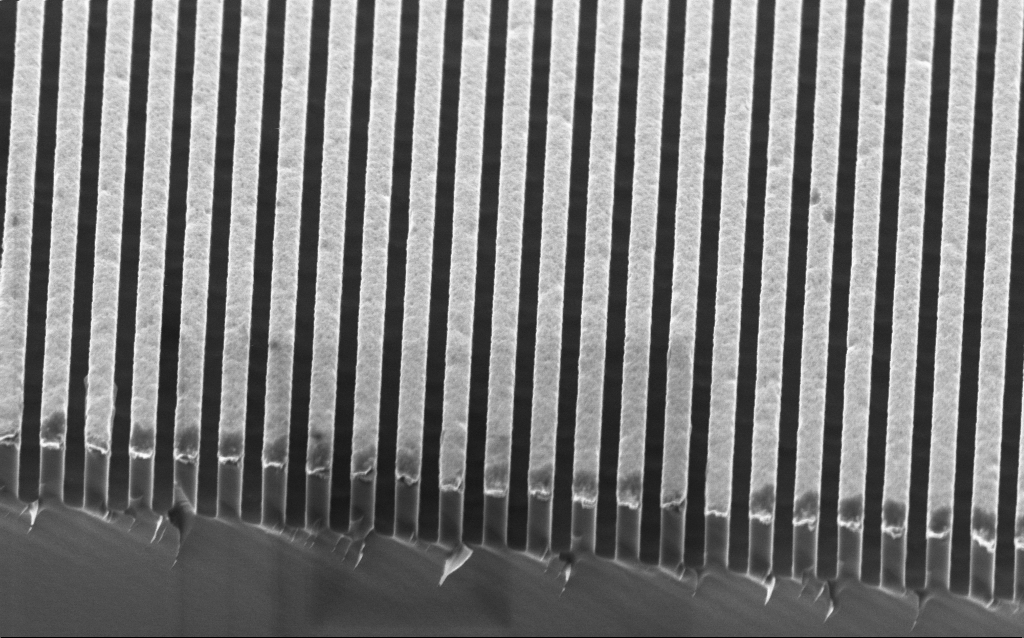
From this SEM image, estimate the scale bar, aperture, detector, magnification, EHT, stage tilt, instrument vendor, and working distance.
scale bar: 1000 nm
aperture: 30 µm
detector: InLens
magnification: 32.81 K X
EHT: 2 kV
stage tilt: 45°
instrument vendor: Zeiss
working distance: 2.9 mm